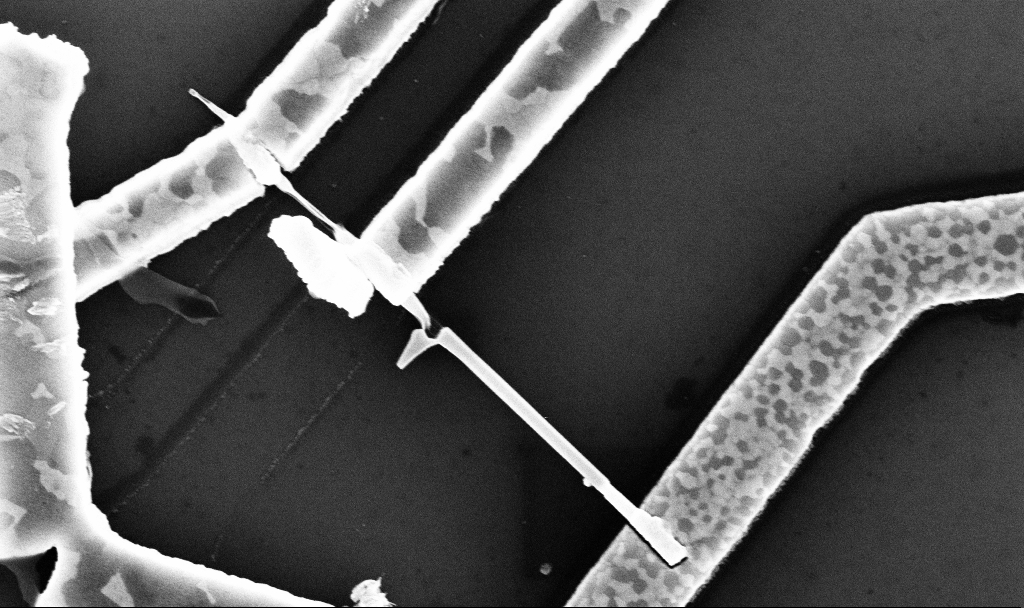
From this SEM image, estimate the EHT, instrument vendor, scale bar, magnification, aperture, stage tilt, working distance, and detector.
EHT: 10 kV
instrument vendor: Zeiss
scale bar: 1000 nm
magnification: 50.39 K X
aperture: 30 µm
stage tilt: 0°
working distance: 7.7 mm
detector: InLens